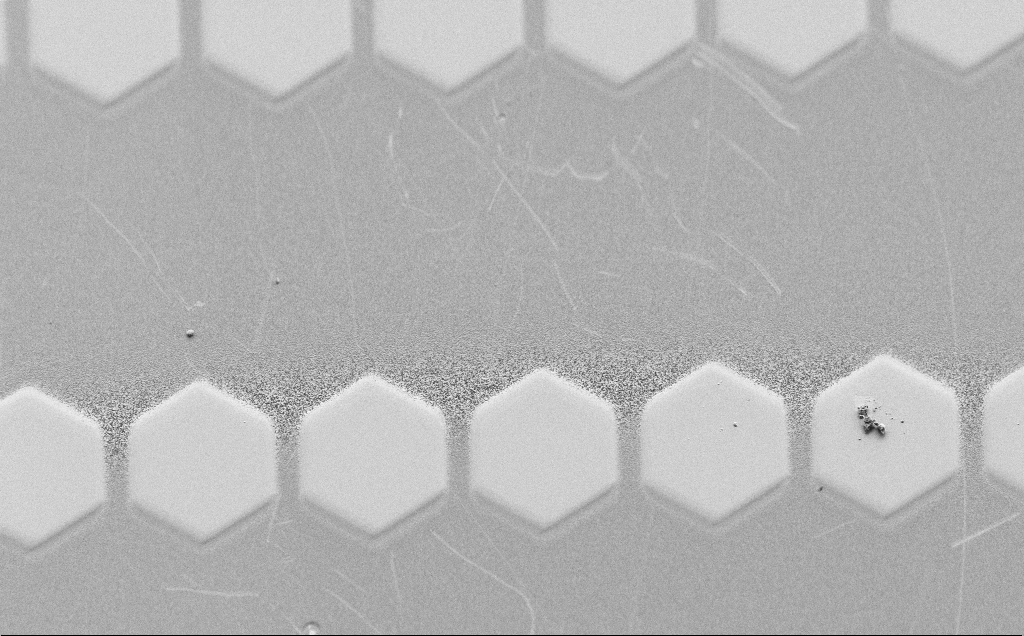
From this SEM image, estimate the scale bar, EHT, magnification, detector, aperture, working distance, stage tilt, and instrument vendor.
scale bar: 20000 nm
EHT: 1.5 kV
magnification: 1.07 K X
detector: SE2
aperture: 30 µm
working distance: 4 mm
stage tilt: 45°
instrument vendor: Zeiss